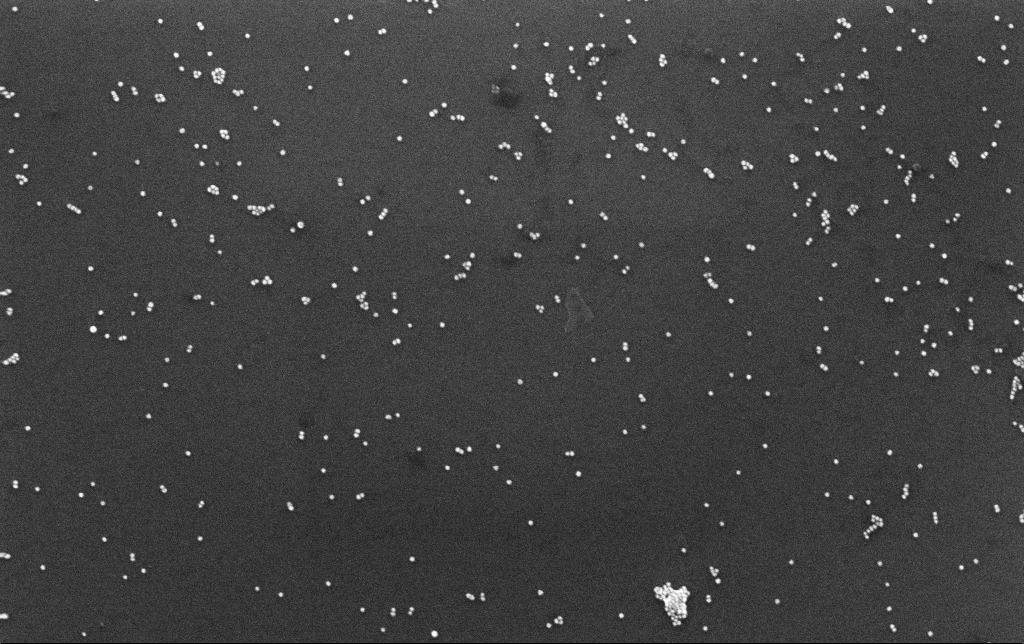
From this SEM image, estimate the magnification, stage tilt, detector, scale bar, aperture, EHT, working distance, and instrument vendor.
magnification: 100 K X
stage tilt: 0°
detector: InLens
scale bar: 200 nm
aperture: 30 µm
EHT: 10 kV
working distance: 3 mm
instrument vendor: Zeiss